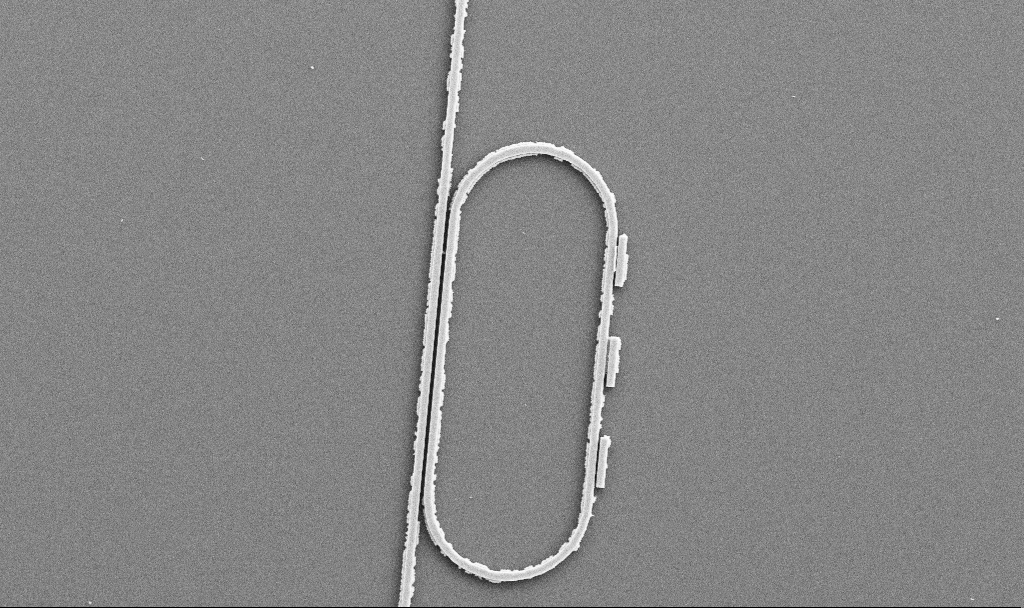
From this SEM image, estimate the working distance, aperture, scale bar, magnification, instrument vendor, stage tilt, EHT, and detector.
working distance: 7.4 mm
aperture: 30 µm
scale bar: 10000 nm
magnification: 6.23 K X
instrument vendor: Zeiss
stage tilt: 0°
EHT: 5 kV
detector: SE2